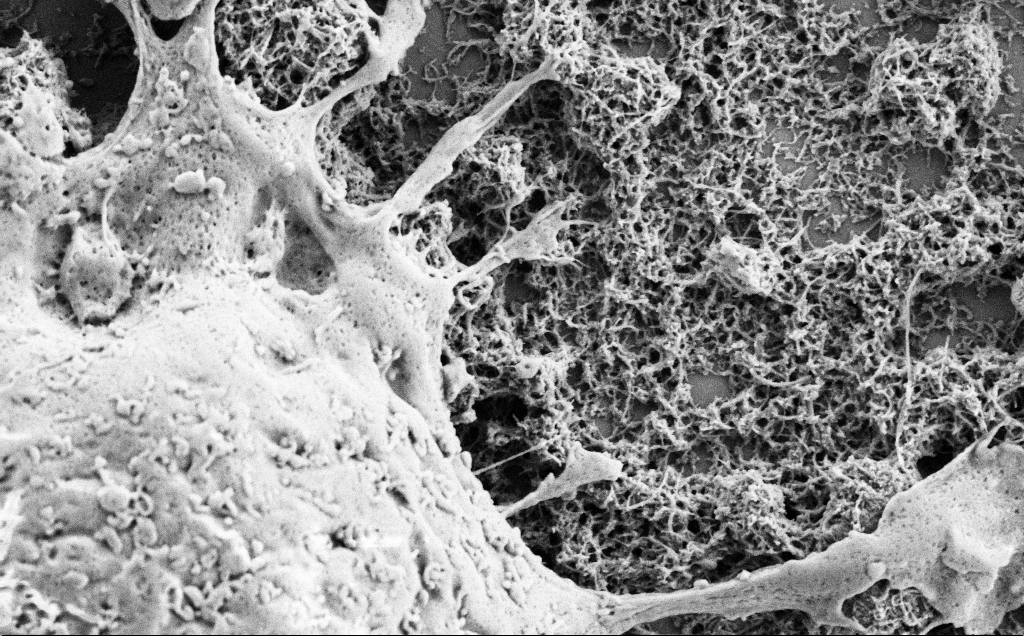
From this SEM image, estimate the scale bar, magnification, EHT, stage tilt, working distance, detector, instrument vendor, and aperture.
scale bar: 1000 nm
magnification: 25 K X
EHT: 2 kV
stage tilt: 0°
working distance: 7 mm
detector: SE2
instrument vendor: Zeiss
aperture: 30 µm